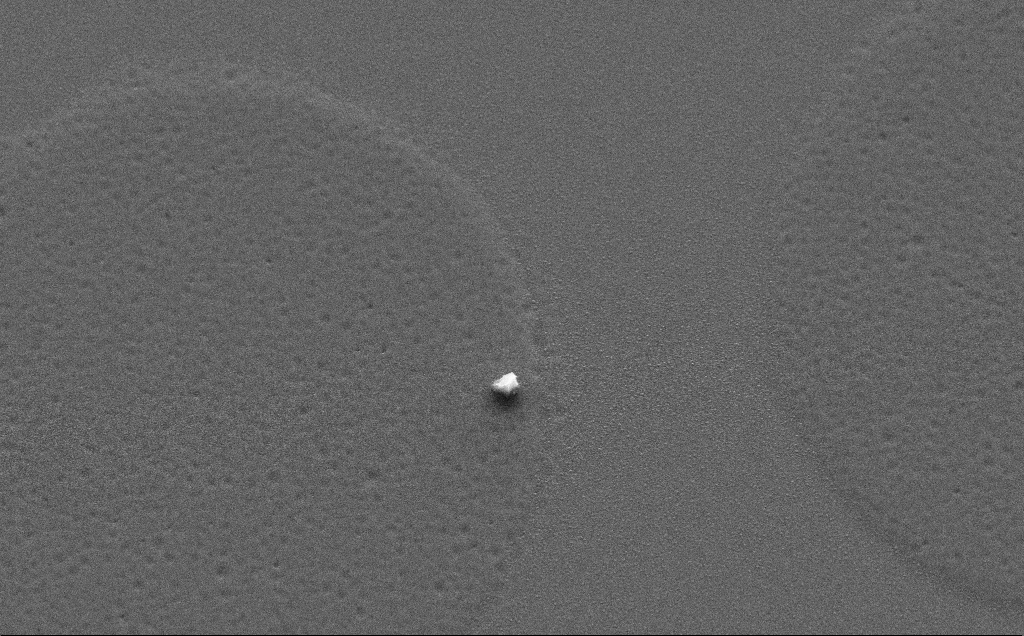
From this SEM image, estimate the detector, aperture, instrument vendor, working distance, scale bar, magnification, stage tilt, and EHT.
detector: SE2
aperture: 30 µm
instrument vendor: Zeiss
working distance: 6 mm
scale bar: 10000 nm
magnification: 6.88 K X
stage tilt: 40.4°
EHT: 10 kV